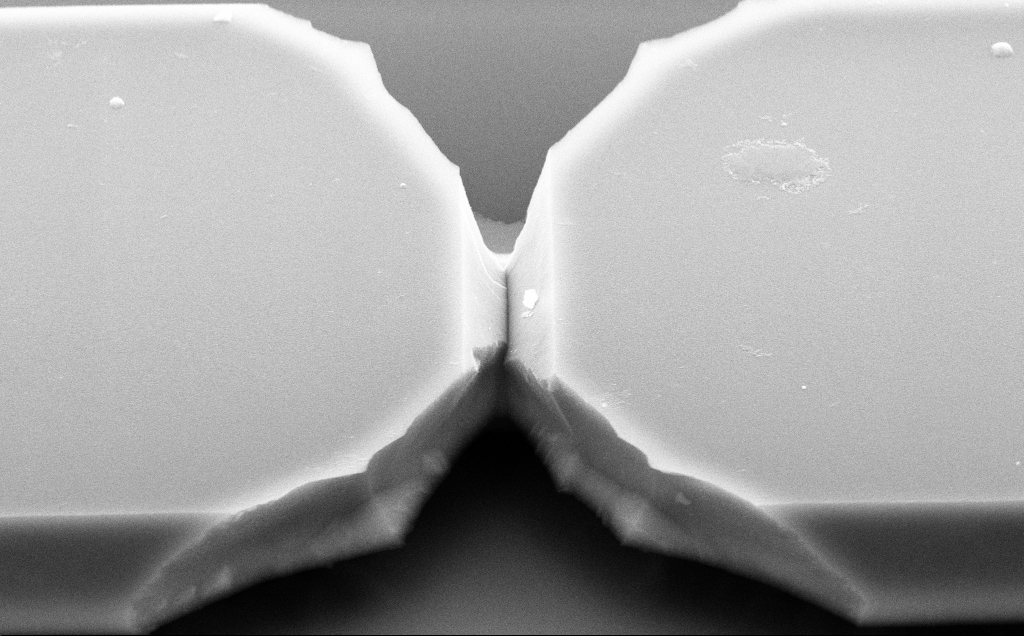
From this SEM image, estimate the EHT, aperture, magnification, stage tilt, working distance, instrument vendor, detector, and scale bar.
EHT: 10 kV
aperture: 30 µm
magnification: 14.99 K X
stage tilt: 50°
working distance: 10 mm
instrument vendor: Zeiss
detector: SE2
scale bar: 2000 nm